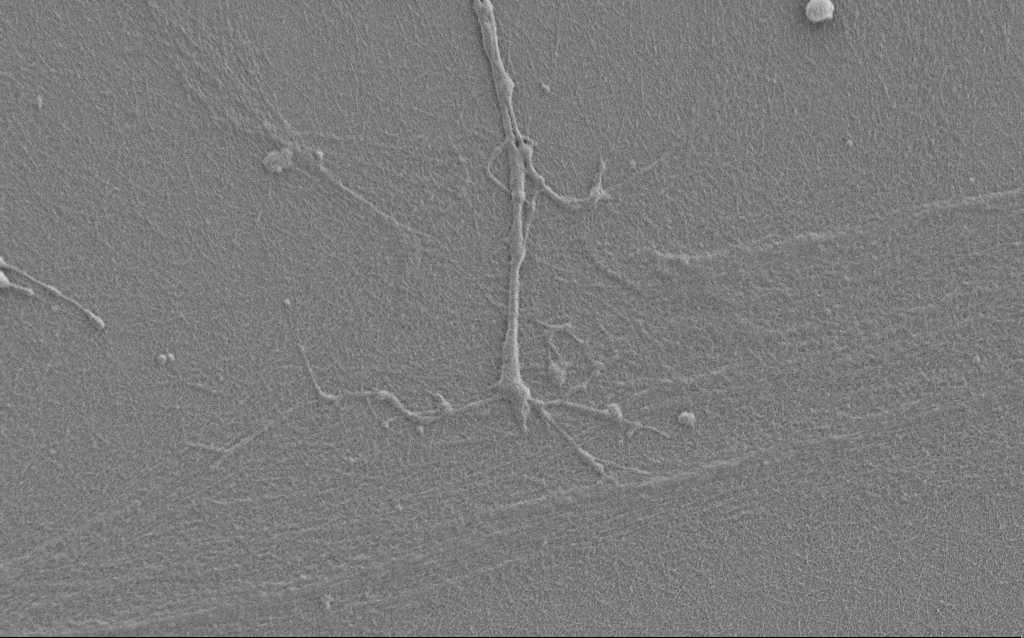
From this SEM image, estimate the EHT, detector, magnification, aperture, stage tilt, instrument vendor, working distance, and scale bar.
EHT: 1 kV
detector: SE2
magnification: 7.5 K X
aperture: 30 µm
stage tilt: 0°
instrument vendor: Zeiss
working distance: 6 mm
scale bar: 2000 nm